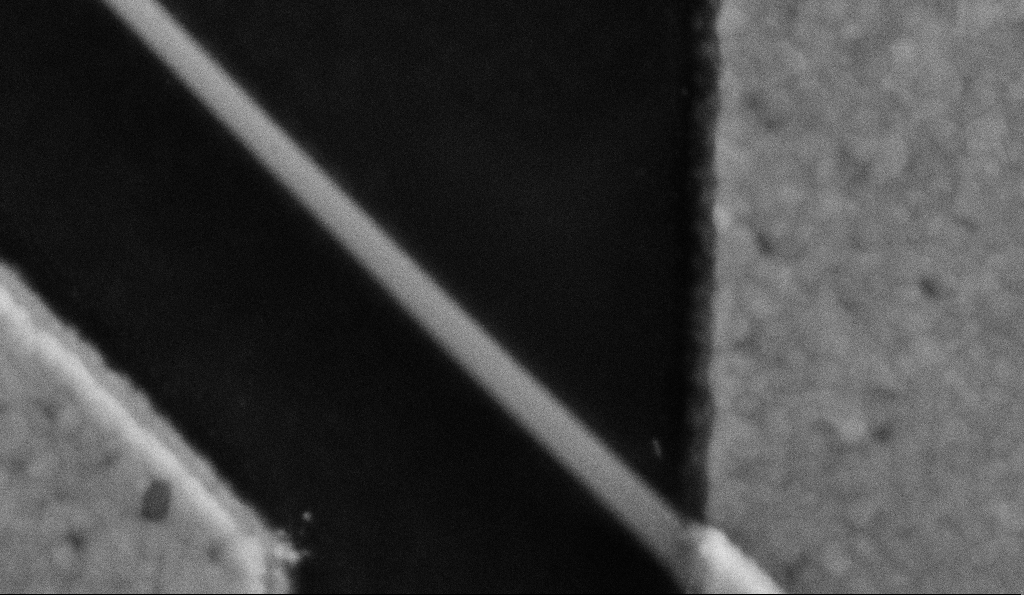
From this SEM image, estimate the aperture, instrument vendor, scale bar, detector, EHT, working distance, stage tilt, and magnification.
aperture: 30 µm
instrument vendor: Zeiss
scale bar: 200 nm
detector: SE2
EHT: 5 kV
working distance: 8.5 mm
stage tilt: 0°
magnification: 200 K X